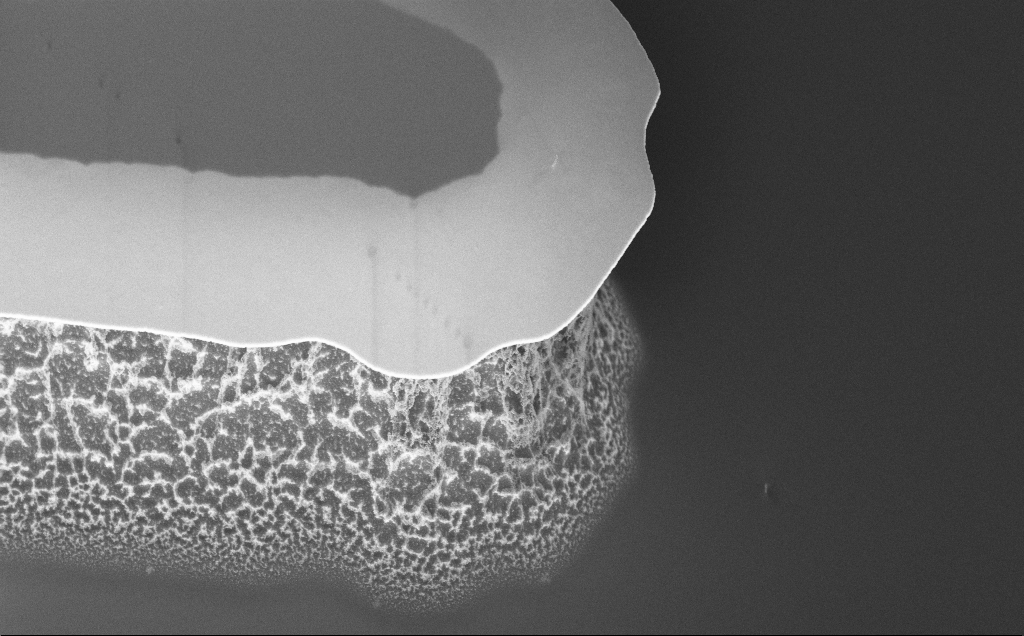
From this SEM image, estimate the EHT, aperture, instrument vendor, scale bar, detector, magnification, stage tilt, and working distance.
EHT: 5 kV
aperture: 30 µm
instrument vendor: Zeiss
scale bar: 2000 nm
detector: InLens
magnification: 8.08 K X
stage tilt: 45°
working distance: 8 mm